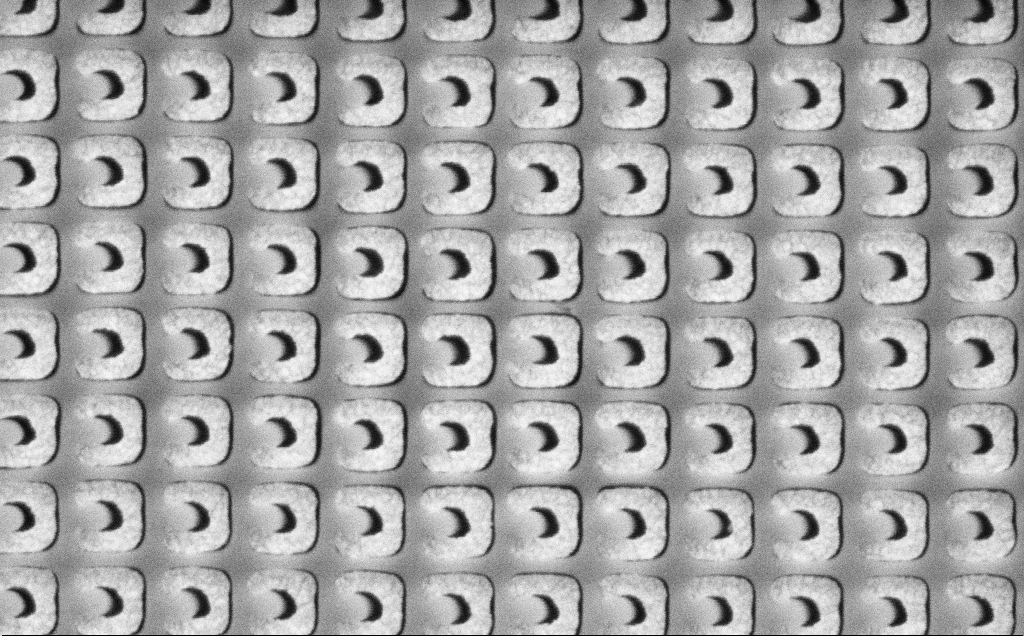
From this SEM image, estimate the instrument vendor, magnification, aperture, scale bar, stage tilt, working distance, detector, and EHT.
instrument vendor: Zeiss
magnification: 68.61 K X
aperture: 30 µm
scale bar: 1000 nm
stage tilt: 0°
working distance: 8.3 mm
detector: SE2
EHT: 3 kV